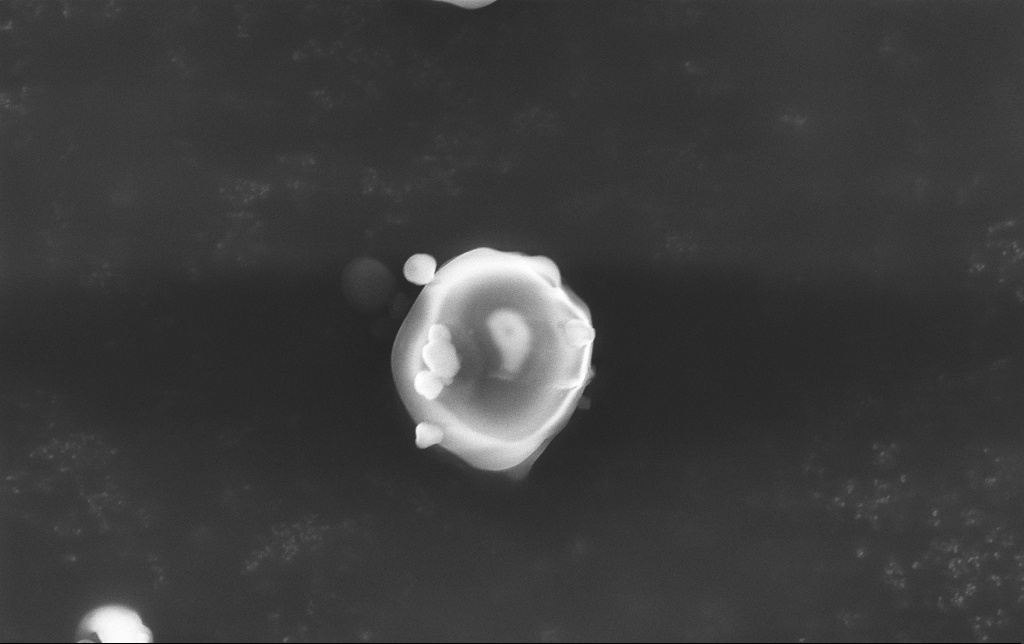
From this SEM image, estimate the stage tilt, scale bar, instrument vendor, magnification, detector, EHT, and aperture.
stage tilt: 0°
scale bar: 2000 nm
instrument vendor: Zeiss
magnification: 10.55 K X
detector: InLens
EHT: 15 kV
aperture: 30 µm